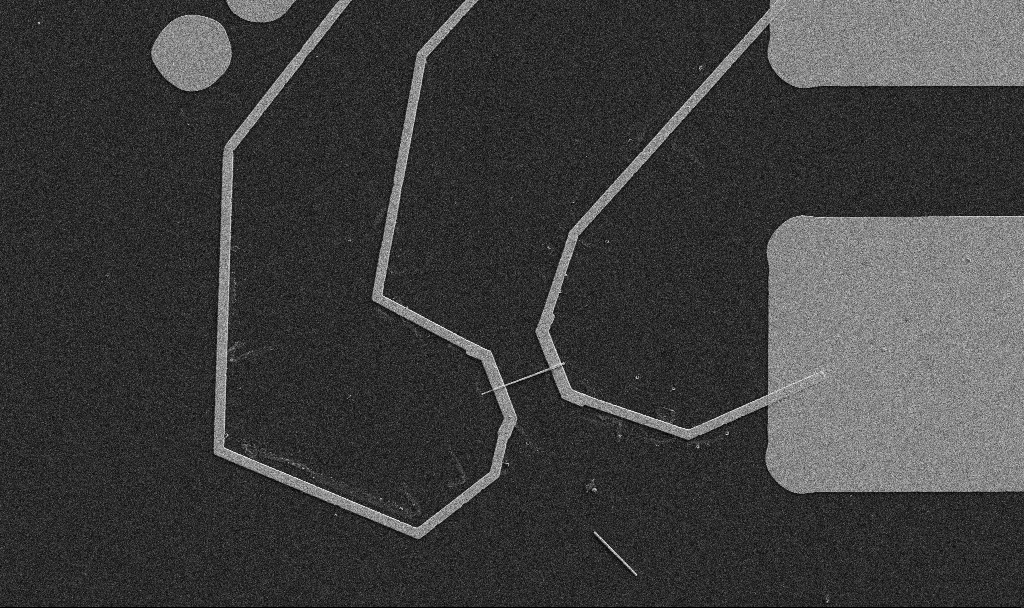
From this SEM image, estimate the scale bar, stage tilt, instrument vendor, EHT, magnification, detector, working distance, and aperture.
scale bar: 10000 nm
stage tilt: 0°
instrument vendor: Zeiss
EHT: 5 kV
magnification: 5 K X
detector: SE2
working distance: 10.7 mm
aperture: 30 µm